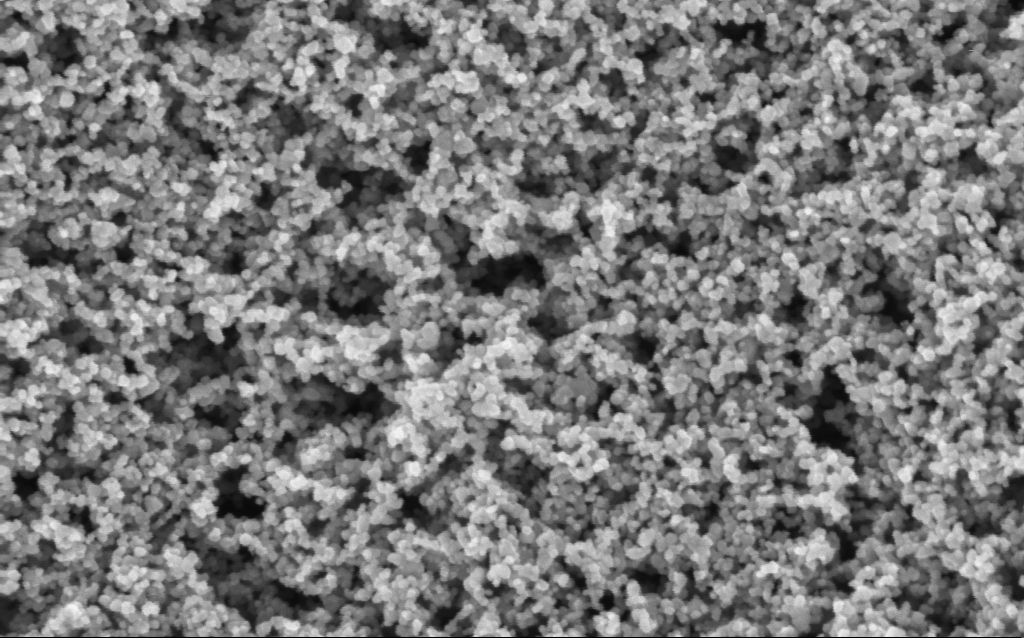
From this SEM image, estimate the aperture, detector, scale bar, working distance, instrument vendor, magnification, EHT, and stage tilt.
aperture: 30 µm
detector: InLens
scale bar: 100 nm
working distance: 7.6 mm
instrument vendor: Zeiss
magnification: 130 K X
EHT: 3 kV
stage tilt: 0°